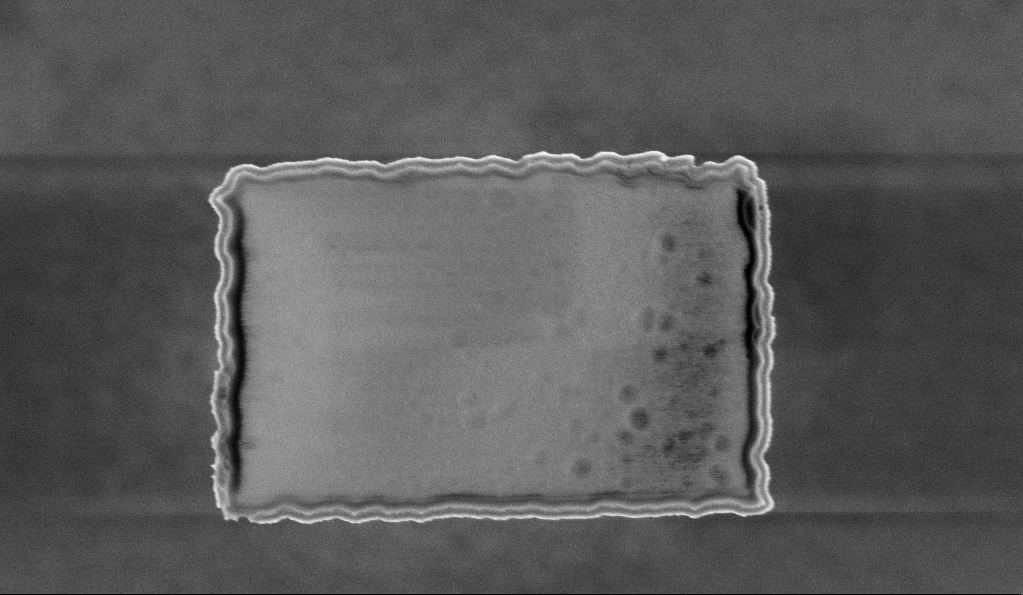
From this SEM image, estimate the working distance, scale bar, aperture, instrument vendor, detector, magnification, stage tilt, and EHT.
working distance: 5.9 mm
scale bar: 1000 nm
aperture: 30 µm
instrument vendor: Zeiss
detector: InLens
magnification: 46.16 K X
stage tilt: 0°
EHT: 3 kV